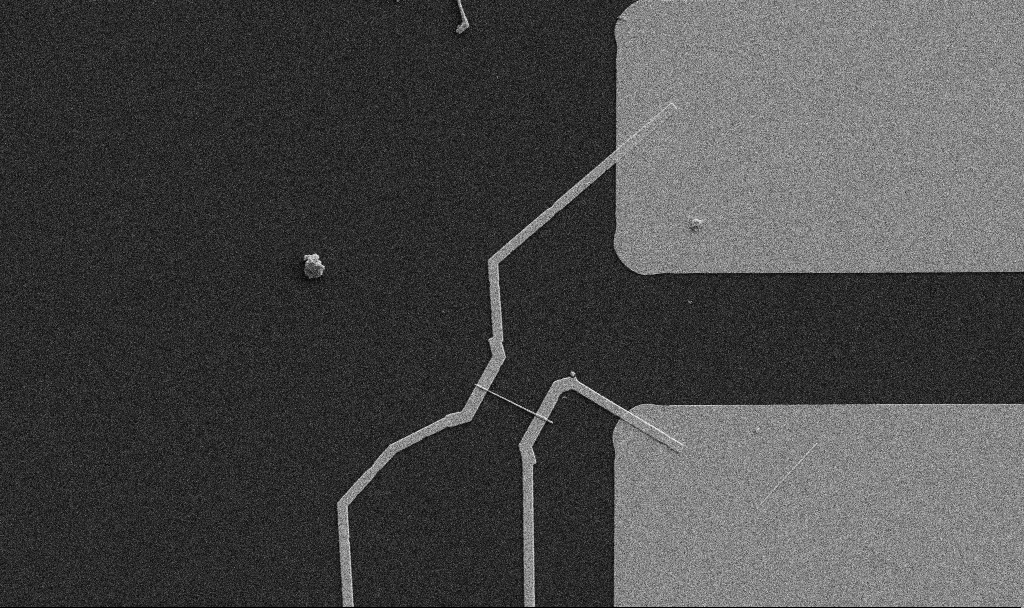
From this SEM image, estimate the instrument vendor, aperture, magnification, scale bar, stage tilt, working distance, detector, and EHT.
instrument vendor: Zeiss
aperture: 30 µm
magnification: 5 K X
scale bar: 10000 nm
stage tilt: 0°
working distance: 10.7 mm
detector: SE2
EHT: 5 kV